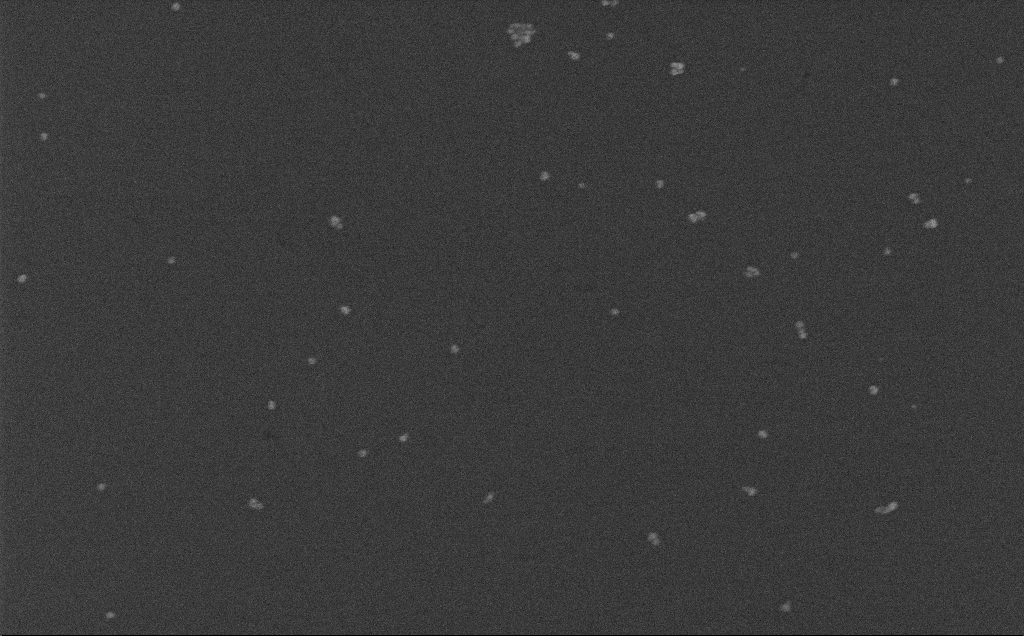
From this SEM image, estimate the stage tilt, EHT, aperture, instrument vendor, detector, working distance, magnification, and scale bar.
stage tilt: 0°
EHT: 10 kV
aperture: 30 µm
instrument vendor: Zeiss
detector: InLens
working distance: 3.7 mm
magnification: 100 K X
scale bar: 200 nm